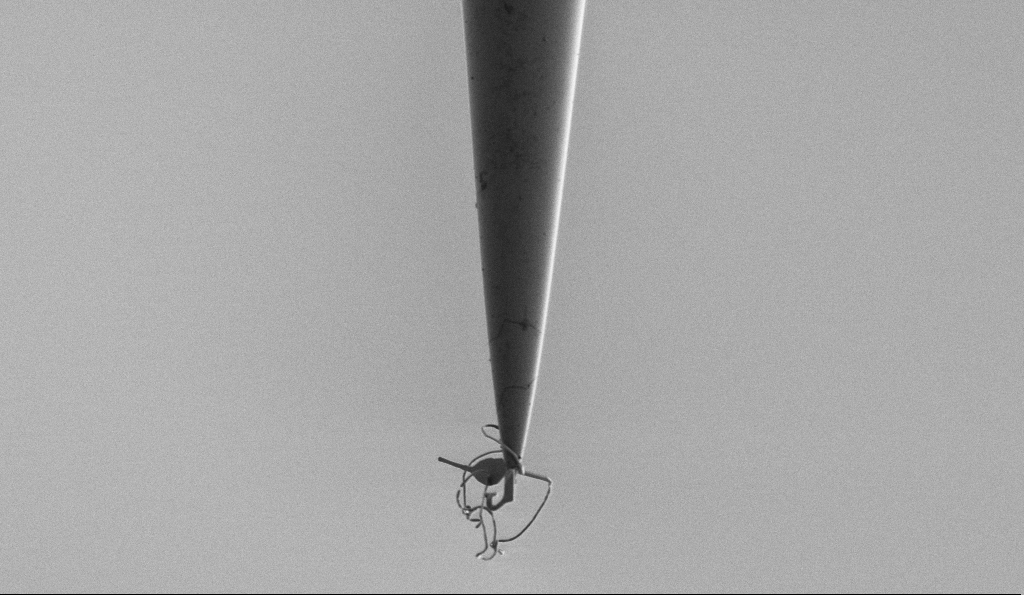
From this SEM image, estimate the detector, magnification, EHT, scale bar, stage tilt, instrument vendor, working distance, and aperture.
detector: SE2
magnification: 2.5 K X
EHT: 1 kV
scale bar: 10000 nm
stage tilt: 0°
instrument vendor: Zeiss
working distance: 6.6 mm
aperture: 30 µm